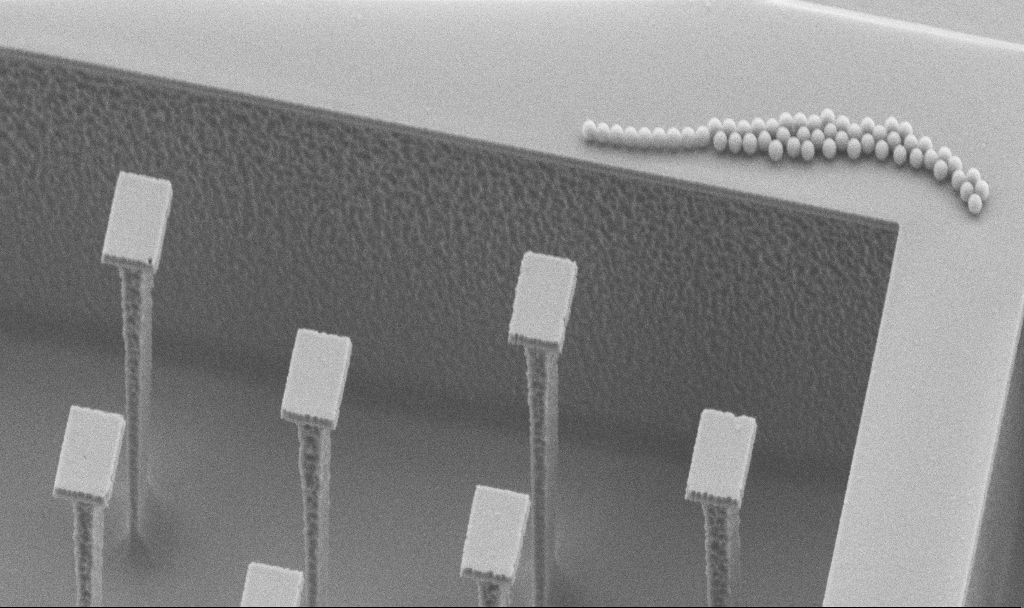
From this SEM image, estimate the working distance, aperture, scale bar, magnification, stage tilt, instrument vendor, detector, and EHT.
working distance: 5.9 mm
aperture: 30 µm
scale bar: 2000 nm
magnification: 7.29 K X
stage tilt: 45°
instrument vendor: Zeiss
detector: SE2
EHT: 5 kV